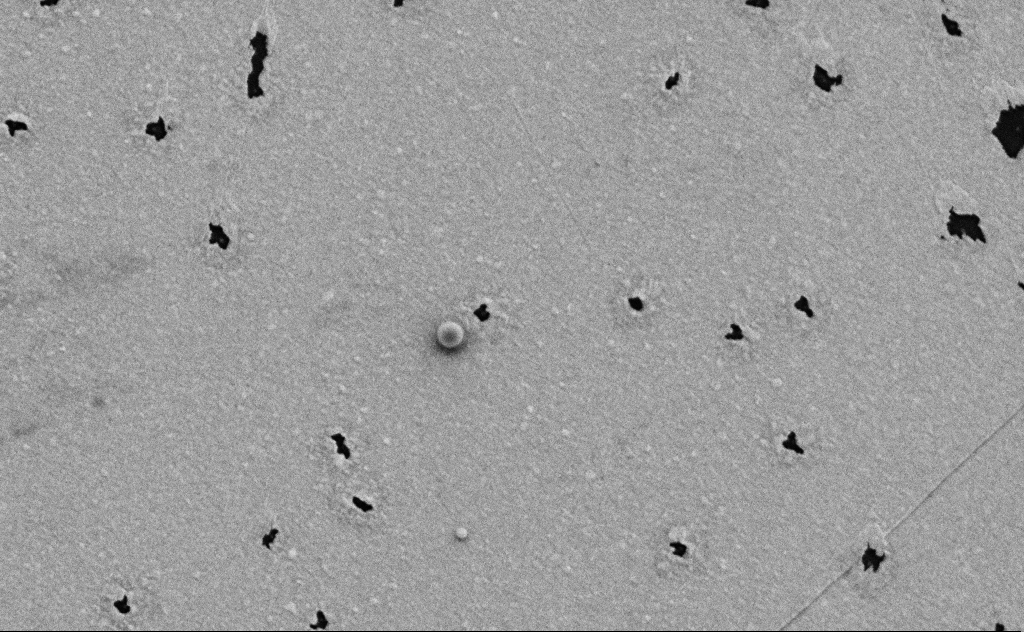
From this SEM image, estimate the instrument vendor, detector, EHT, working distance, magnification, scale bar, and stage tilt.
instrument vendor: Zeiss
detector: SE2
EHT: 3 kV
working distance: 10 mm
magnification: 31.36 K X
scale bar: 1000 nm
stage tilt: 0°